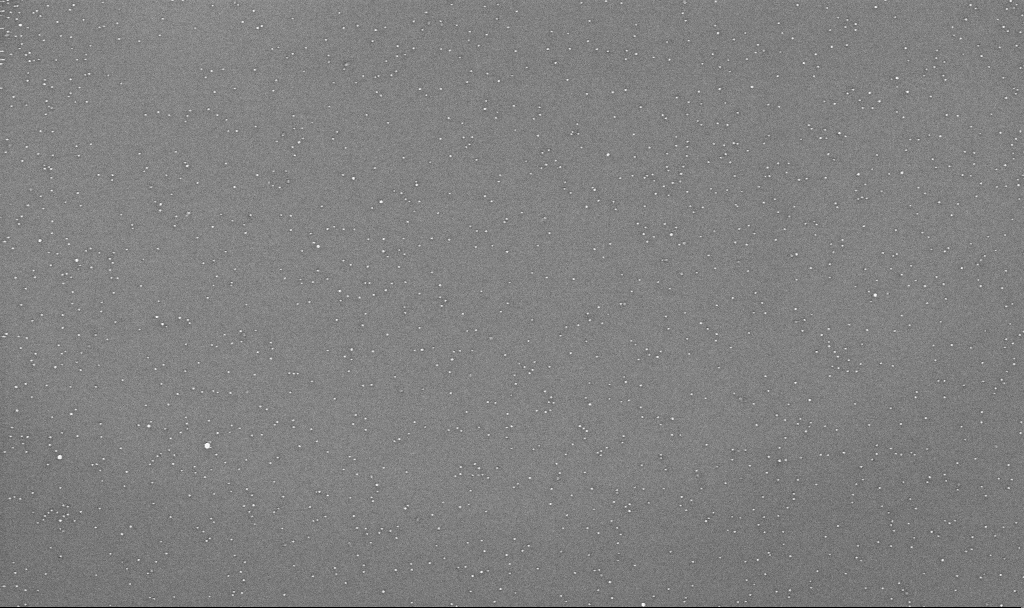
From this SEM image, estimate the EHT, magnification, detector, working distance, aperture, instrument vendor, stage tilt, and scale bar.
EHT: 10 kV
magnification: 70 K X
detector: InLens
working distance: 3.3 mm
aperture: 30 µm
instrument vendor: Zeiss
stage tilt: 0°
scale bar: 1000 nm